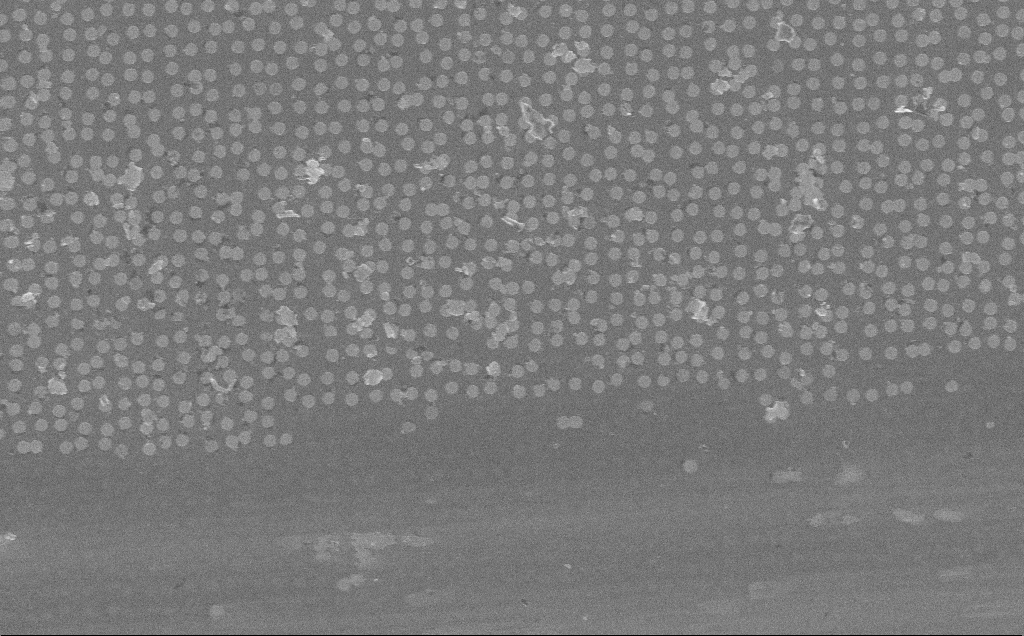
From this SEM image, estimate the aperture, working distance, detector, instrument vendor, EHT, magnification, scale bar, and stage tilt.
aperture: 30 µm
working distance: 7 mm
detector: InLens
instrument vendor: Zeiss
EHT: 10 kV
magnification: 19.03 K X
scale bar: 2000 nm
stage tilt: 0°